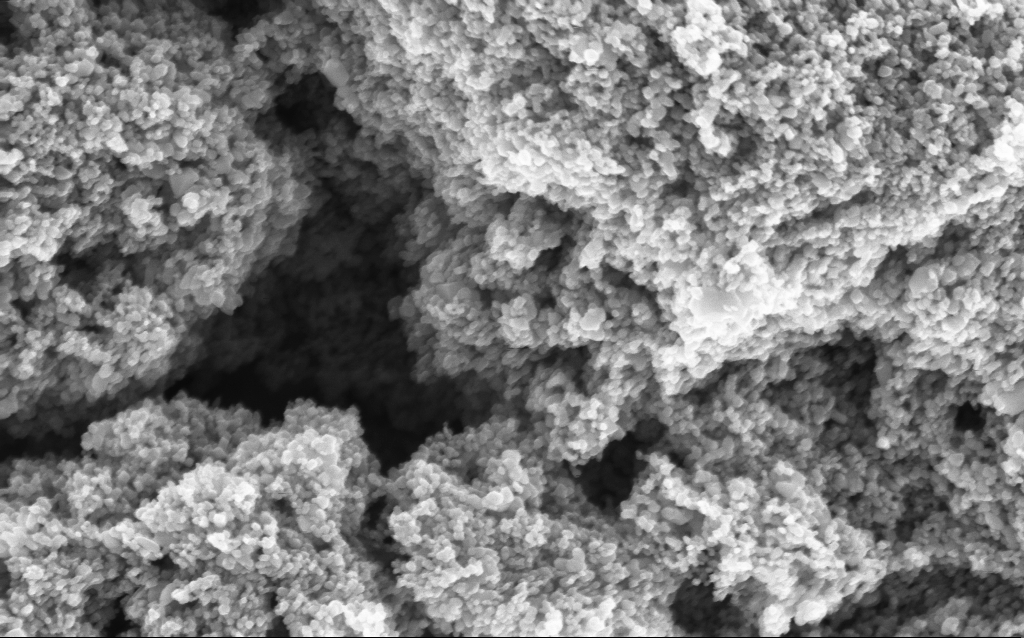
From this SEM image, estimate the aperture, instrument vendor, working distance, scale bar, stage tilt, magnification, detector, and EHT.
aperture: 30 µm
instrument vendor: Zeiss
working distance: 4.7 mm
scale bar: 200 nm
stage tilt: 0°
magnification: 114.65 K X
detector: InLens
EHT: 5 kV